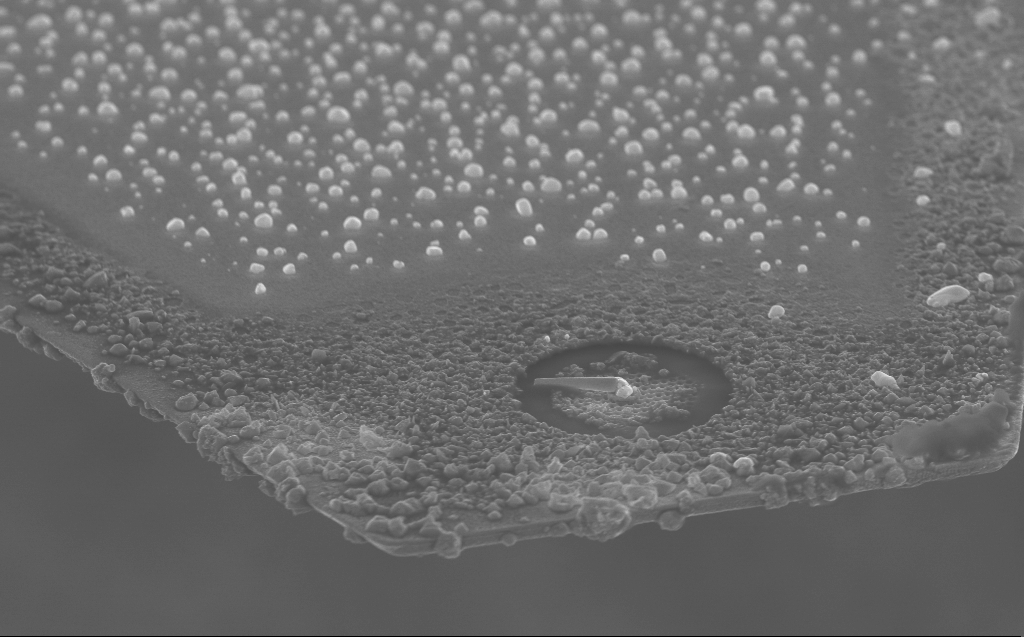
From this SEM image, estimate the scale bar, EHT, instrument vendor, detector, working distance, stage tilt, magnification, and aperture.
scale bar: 1000 nm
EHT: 10 kV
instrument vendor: Zeiss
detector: InLens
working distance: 5 mm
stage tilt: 60°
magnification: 13.78 K X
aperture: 30 µm